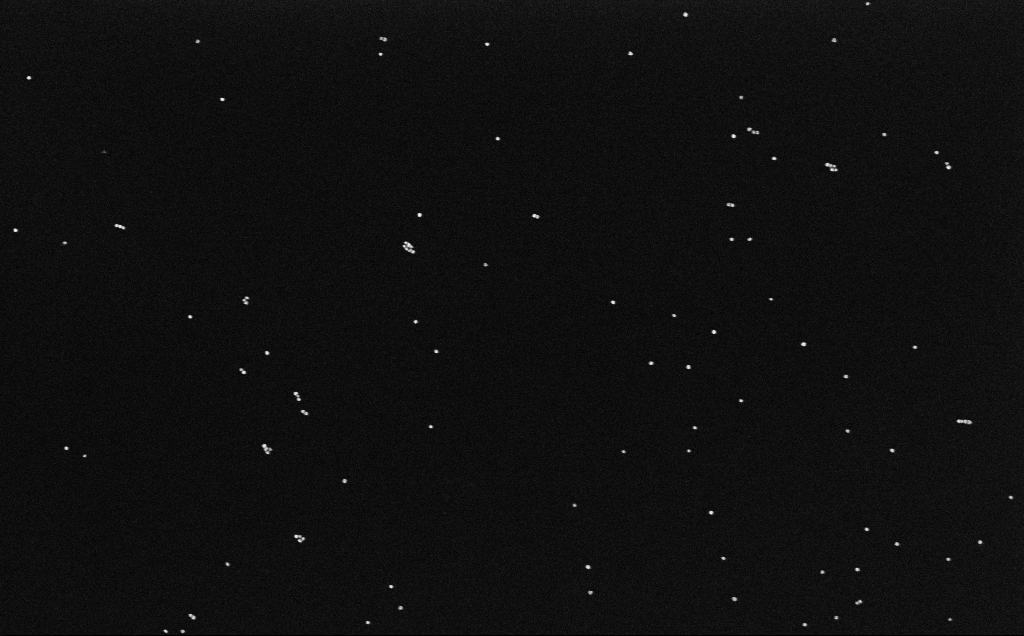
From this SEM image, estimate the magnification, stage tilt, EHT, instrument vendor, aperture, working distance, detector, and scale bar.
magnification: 100 K X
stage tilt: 0°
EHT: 10 kV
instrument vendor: Zeiss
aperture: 30 µm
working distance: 6.6 mm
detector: InLens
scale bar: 200 nm